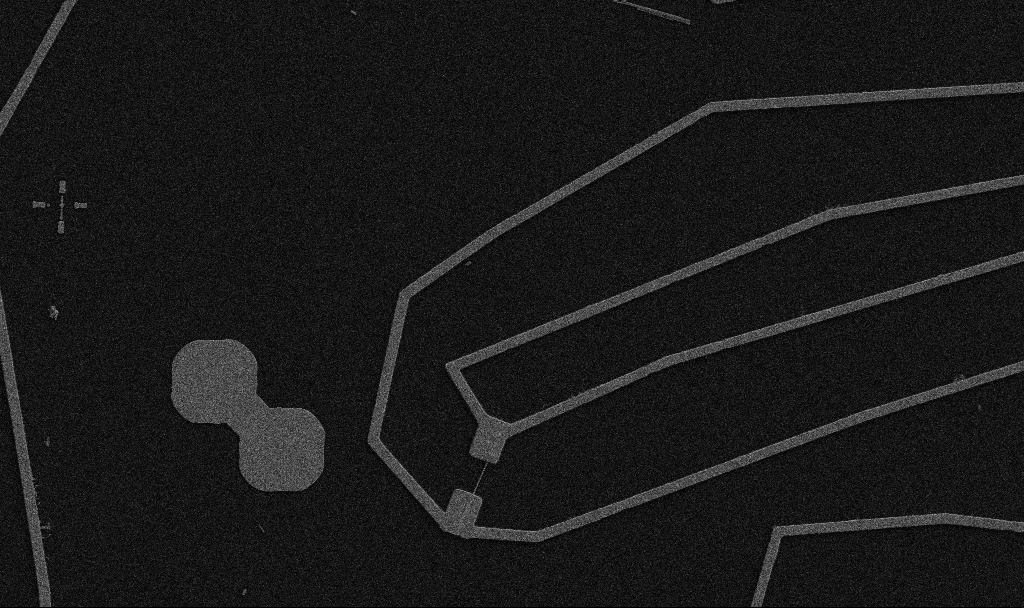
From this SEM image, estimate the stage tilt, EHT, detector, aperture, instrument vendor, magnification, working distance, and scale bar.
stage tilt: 0°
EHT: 5 kV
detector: SE2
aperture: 30 µm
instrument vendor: Zeiss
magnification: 5 K X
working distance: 10.7 mm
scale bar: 10000 nm